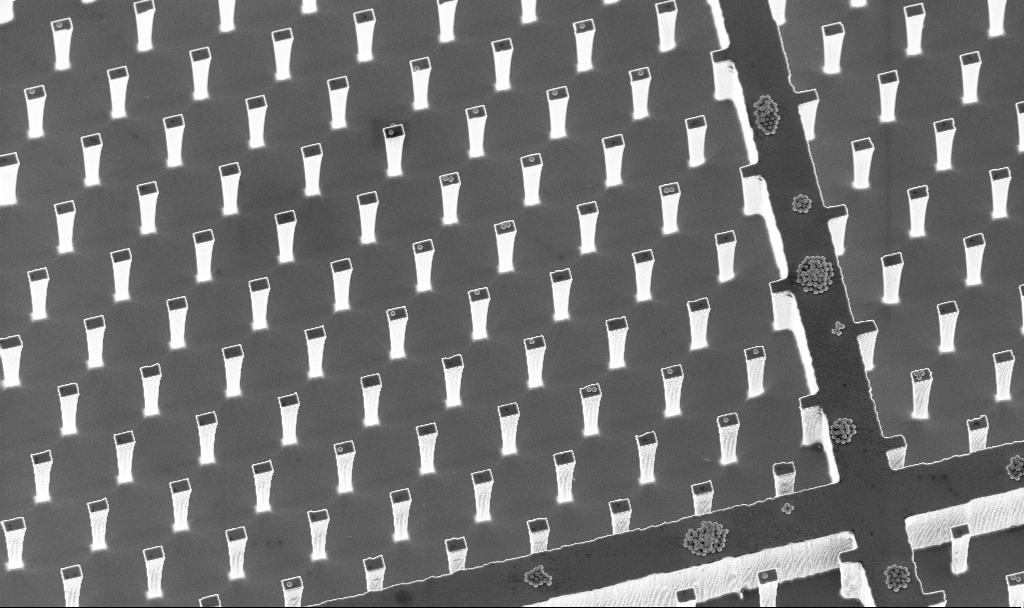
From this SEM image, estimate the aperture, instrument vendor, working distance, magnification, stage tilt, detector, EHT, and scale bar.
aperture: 30 µm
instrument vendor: Zeiss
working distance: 4.7 mm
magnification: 2.59 K X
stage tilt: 20°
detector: InLens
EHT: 5 kV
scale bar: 20000 nm